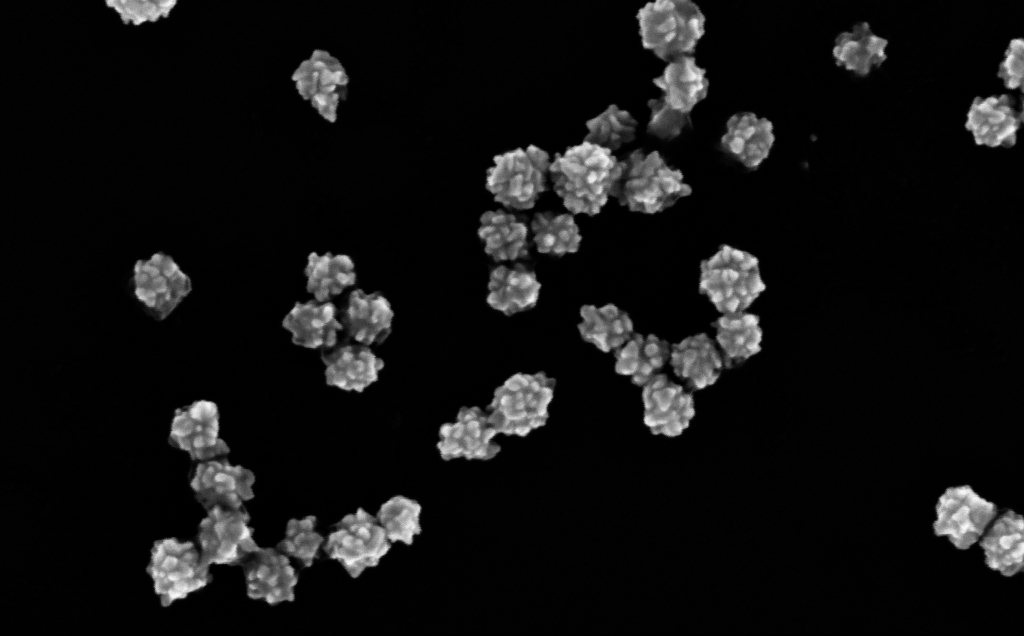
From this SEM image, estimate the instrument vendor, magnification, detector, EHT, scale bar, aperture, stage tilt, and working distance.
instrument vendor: Zeiss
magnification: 317.3 K X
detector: InLens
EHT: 10 kV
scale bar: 100 nm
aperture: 30 µm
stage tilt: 0°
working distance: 3 mm